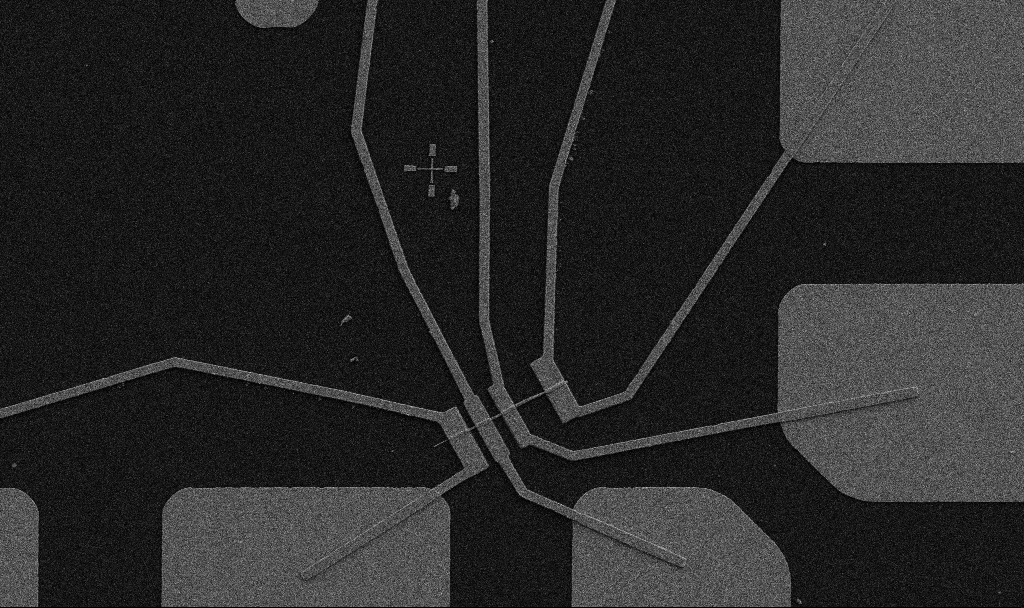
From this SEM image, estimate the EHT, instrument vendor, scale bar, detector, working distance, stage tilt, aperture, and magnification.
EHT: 5 kV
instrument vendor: Zeiss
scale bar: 10000 nm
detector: SE2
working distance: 10.7 mm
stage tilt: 0°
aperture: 30 µm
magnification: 5 K X